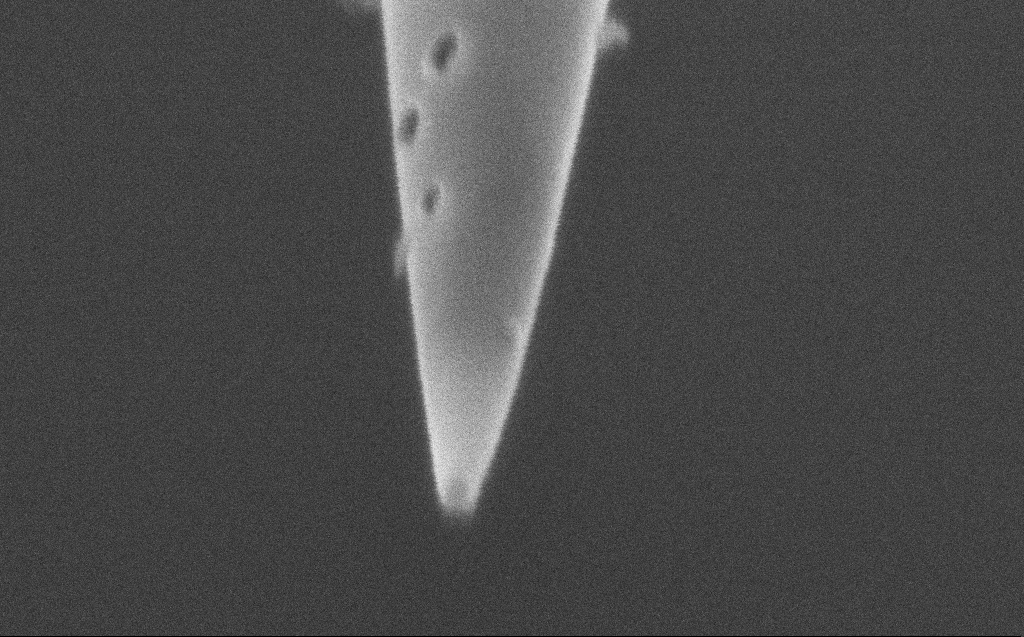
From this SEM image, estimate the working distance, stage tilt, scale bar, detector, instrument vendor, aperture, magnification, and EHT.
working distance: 3 mm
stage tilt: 45.1°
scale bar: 100 nm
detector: InLens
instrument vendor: Zeiss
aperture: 20 µm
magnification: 207.83 K X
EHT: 1.5 kV